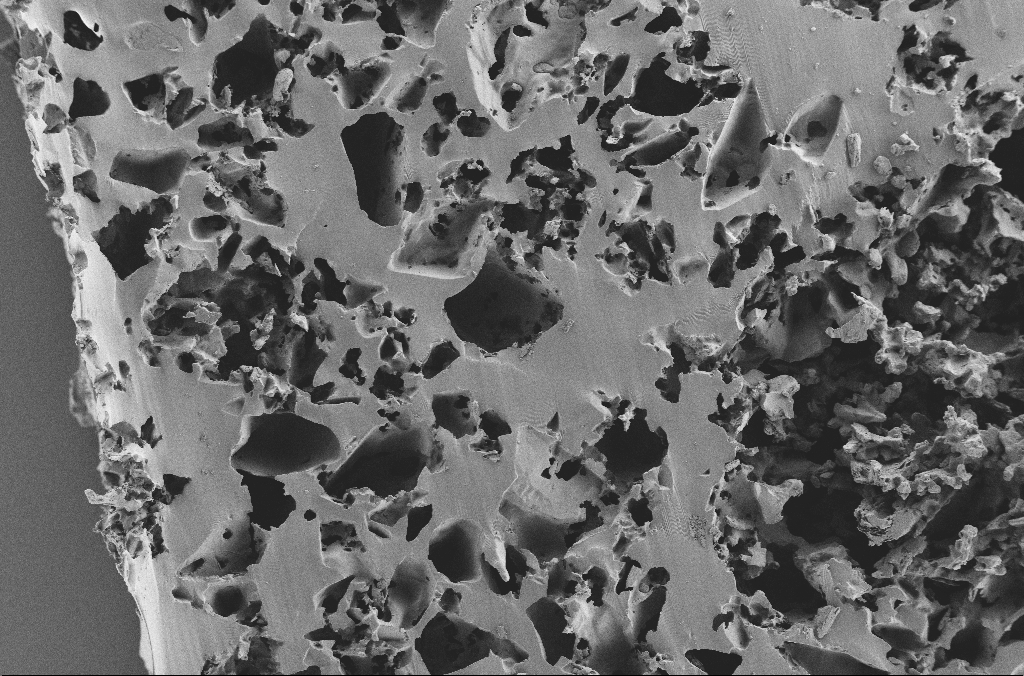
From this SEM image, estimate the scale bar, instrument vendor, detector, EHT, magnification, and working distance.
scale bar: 100000 nm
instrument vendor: Zeiss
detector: SE2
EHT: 2 kV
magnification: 0.25 K X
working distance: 3.1 mm